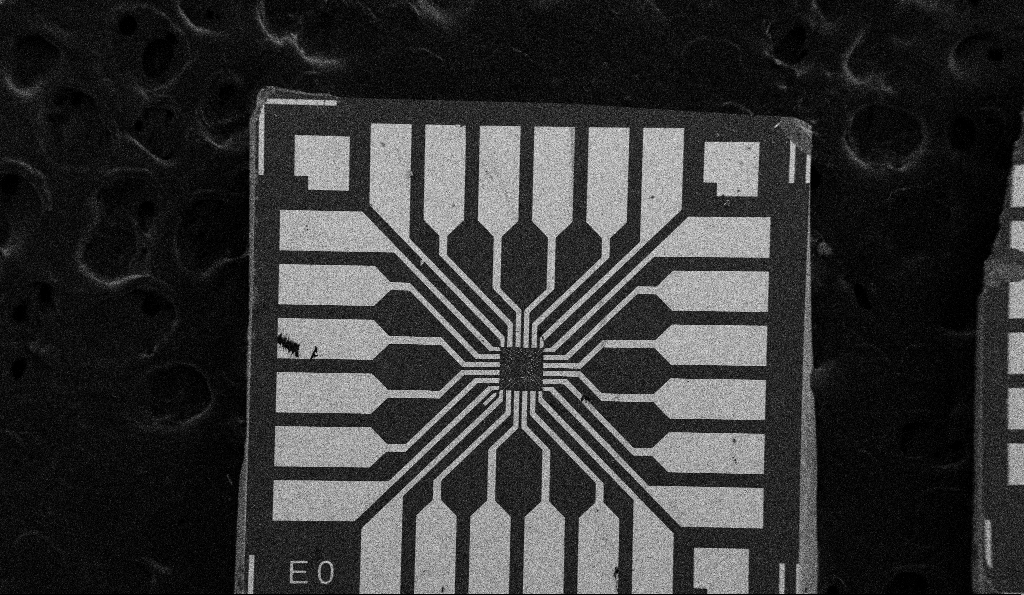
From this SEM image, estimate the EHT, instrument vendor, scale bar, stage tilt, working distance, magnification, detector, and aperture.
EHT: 5 kV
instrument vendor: Zeiss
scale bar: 200000 nm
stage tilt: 0°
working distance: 9.5 mm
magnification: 0.1 K X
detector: SE2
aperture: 30 µm